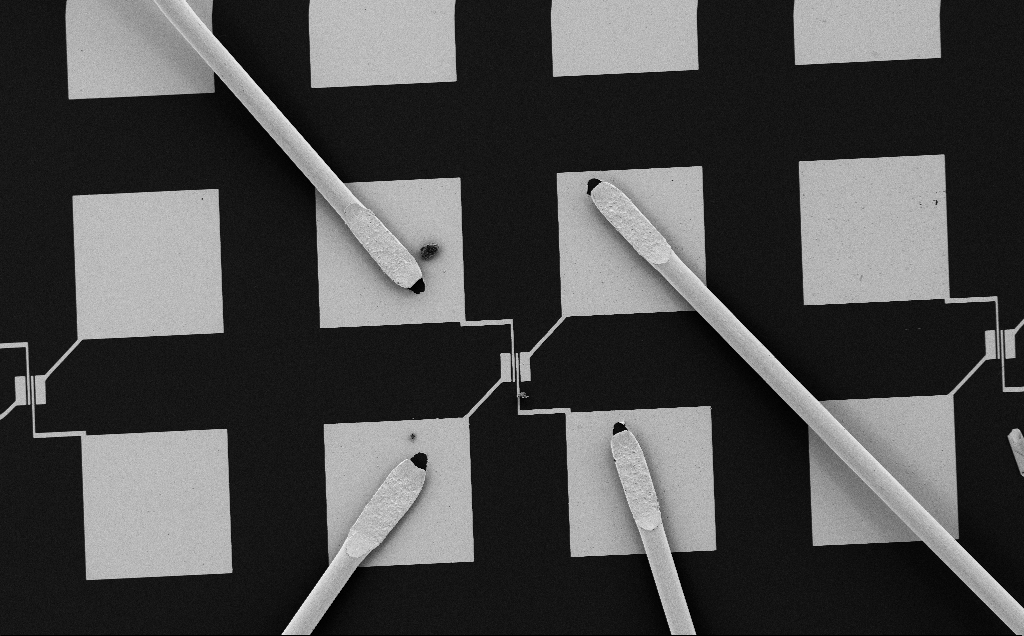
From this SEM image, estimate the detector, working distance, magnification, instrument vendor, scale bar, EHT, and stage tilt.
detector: SE2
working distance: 10 mm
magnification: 0.357 K X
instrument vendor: Zeiss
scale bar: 100000 nm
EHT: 5 kV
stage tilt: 0°